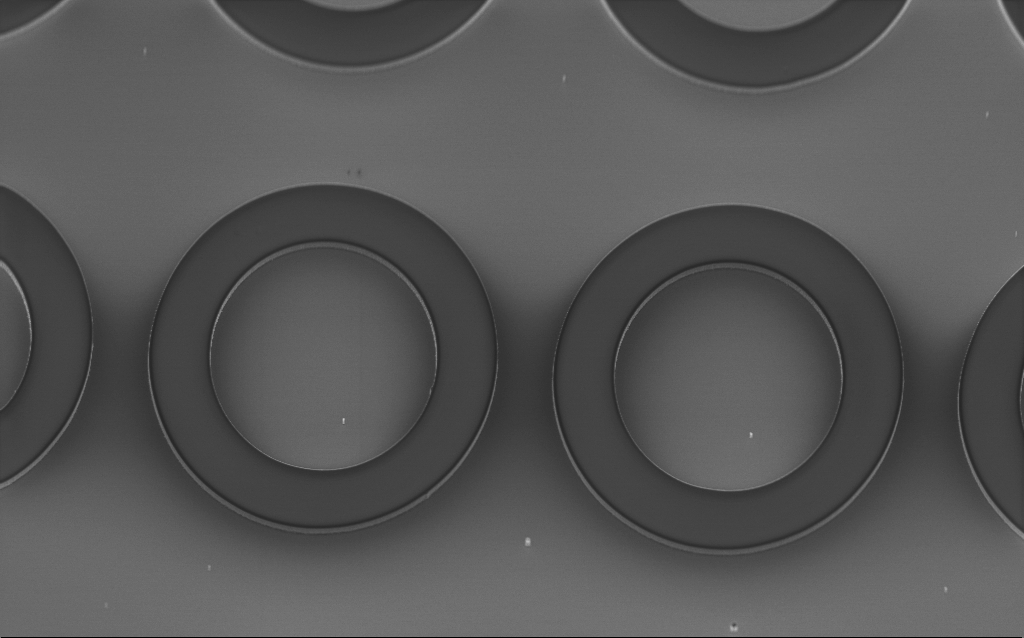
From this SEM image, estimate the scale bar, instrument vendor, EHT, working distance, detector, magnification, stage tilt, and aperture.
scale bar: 20000 nm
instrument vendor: Zeiss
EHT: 2 kV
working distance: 3.4 mm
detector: InLens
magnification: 2.15 K X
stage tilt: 45°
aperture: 30 µm